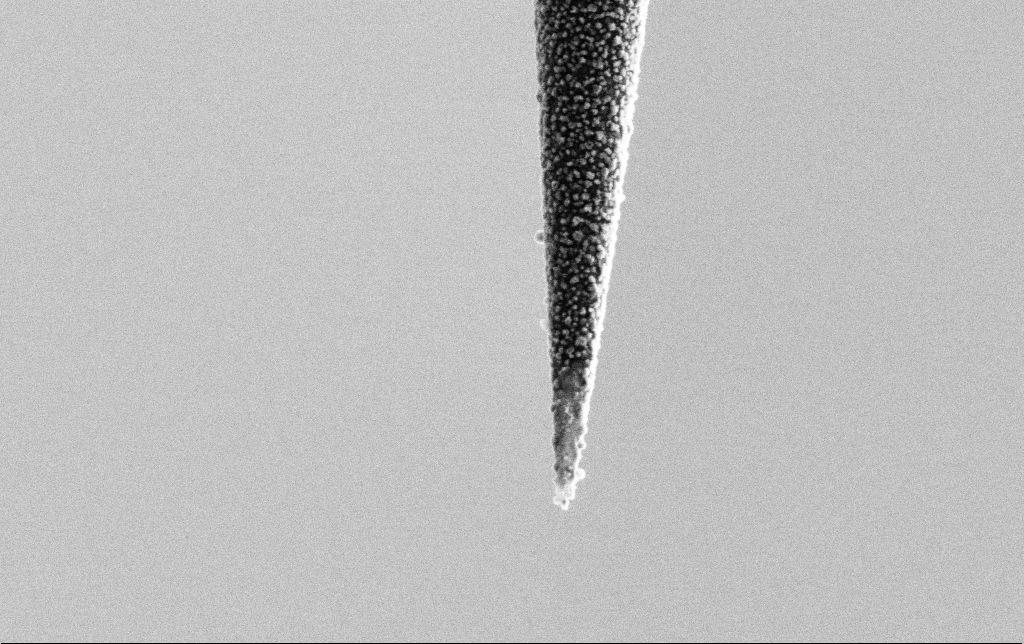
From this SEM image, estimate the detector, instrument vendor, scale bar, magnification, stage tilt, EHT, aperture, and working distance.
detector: SE2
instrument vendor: Zeiss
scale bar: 1000 nm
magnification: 50 K X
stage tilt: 0°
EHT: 2 kV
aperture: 30 µm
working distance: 5.9 mm